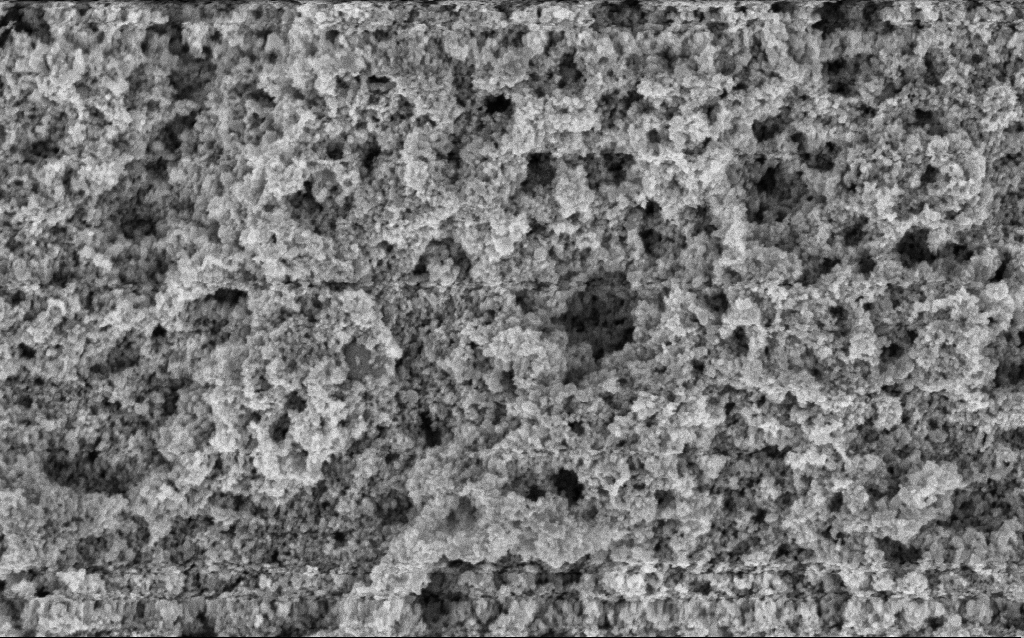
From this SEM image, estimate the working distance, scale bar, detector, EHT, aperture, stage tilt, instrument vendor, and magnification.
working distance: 10 mm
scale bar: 1000 nm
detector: InLens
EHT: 3 kV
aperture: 30 µm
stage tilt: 0°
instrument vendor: Zeiss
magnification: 65.04 K X